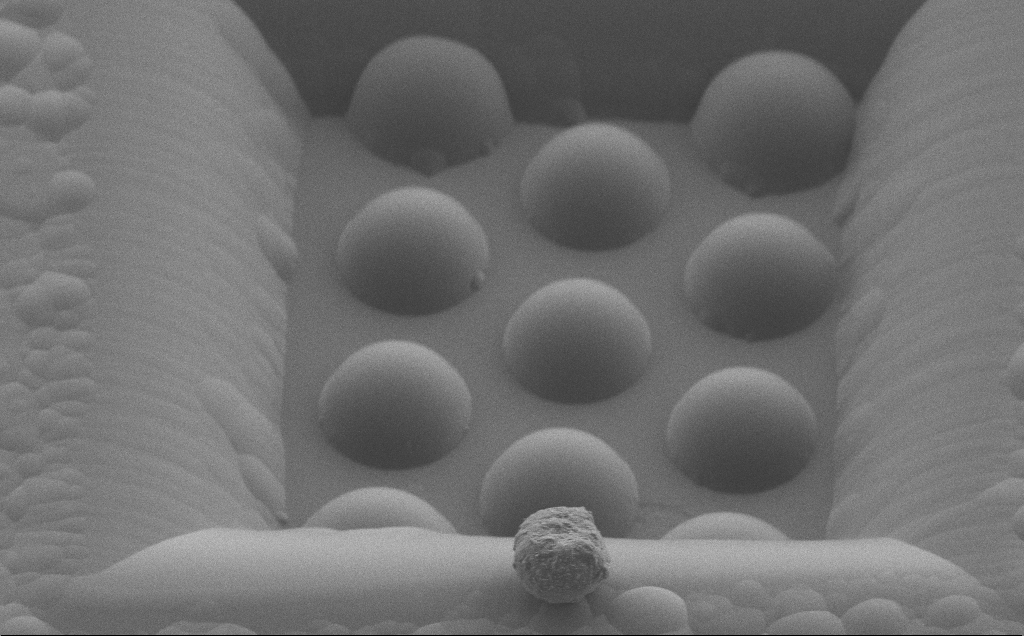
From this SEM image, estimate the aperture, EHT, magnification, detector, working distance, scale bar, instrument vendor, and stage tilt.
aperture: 30 µm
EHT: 1.3 kV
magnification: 3.53 K X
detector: SE2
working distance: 6 mm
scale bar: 20000 nm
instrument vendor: Zeiss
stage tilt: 38.2°